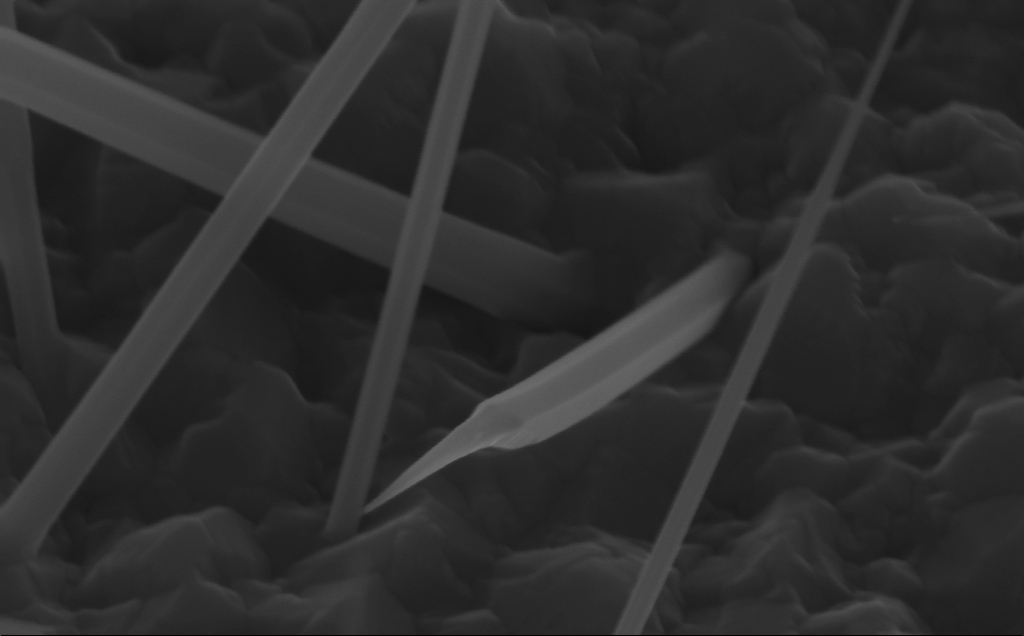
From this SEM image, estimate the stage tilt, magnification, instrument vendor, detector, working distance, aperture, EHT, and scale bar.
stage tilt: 45°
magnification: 175.08 K X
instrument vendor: Zeiss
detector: InLens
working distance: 5 mm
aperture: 30 µm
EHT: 10 kV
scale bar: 200 nm